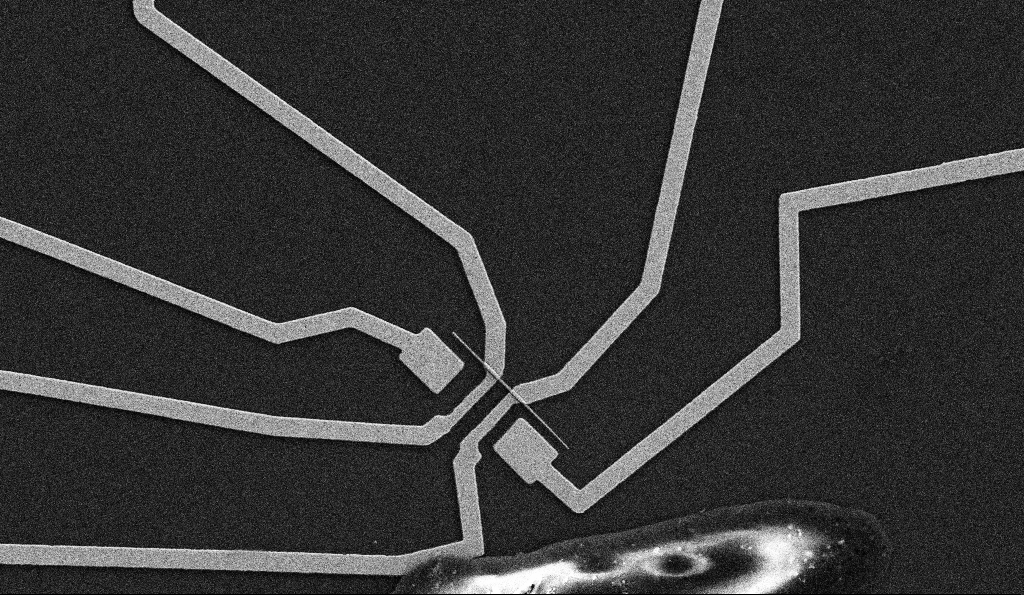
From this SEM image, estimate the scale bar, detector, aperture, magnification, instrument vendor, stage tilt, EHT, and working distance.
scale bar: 2000 nm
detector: SE2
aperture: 30 µm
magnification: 10 K X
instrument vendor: Zeiss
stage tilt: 0°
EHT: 5 kV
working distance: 9.6 mm